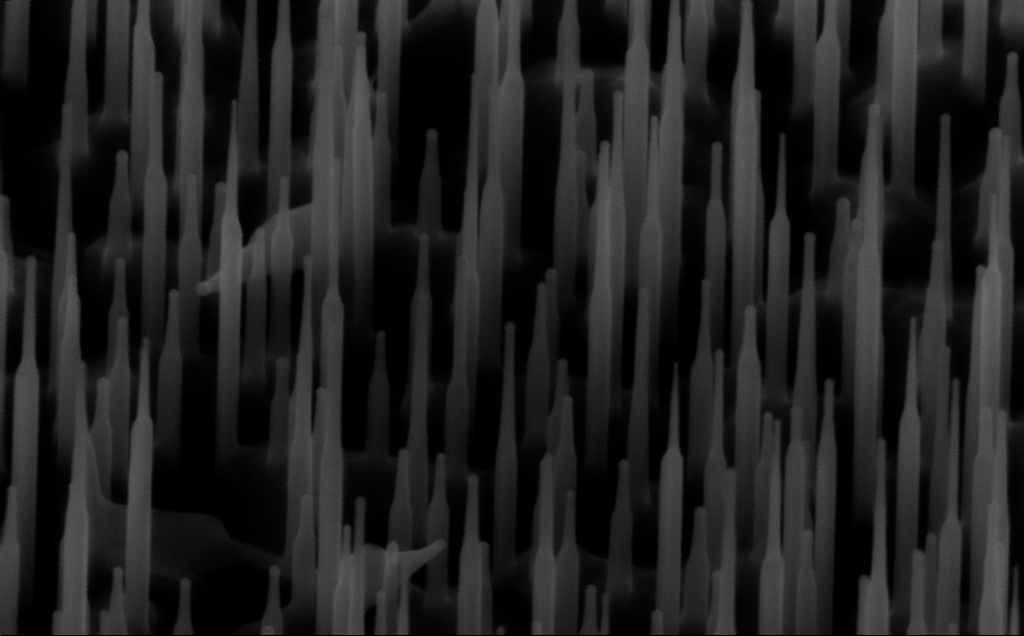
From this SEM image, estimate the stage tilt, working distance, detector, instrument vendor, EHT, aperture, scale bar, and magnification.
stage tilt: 45°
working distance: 6 mm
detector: InLens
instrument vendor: Zeiss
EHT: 10 kV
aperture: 30 µm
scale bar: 100 nm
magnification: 150 K X